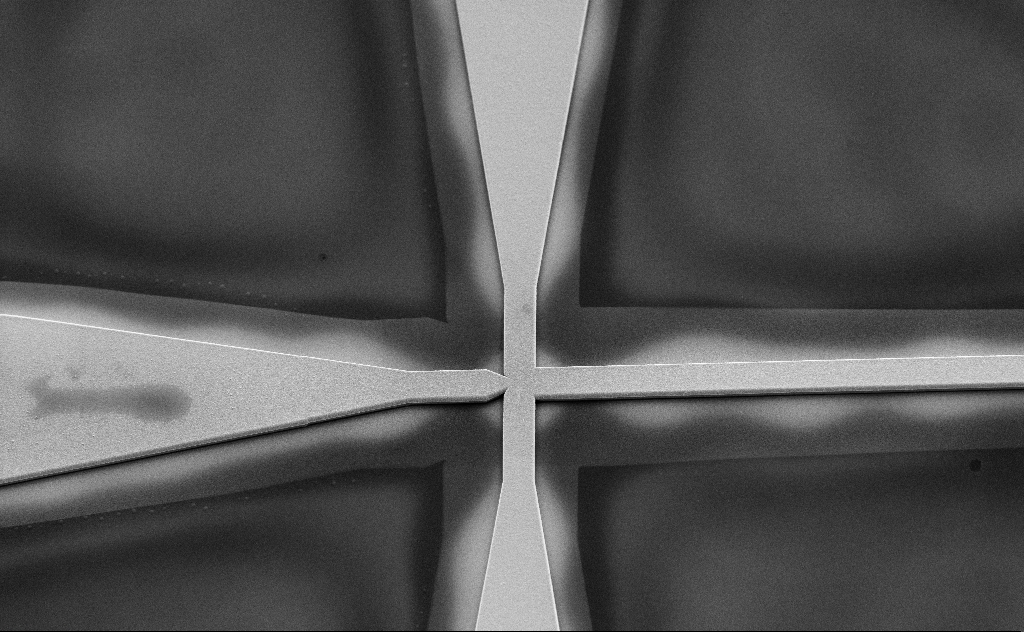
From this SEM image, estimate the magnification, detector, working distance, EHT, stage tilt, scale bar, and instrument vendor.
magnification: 0.939 K X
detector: SE2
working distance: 11 mm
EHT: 10 kV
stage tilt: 45°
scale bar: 20000 nm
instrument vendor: Zeiss